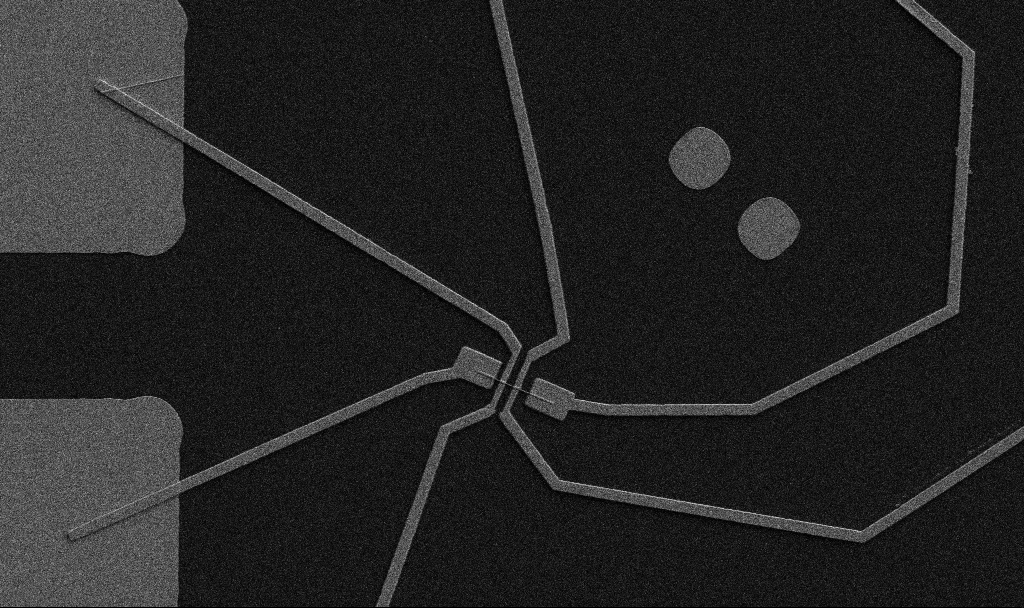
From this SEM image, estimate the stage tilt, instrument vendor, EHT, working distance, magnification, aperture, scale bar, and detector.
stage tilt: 0°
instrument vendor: Zeiss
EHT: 5 kV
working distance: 10.7 mm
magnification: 5 K X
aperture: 30 µm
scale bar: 10000 nm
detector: SE2